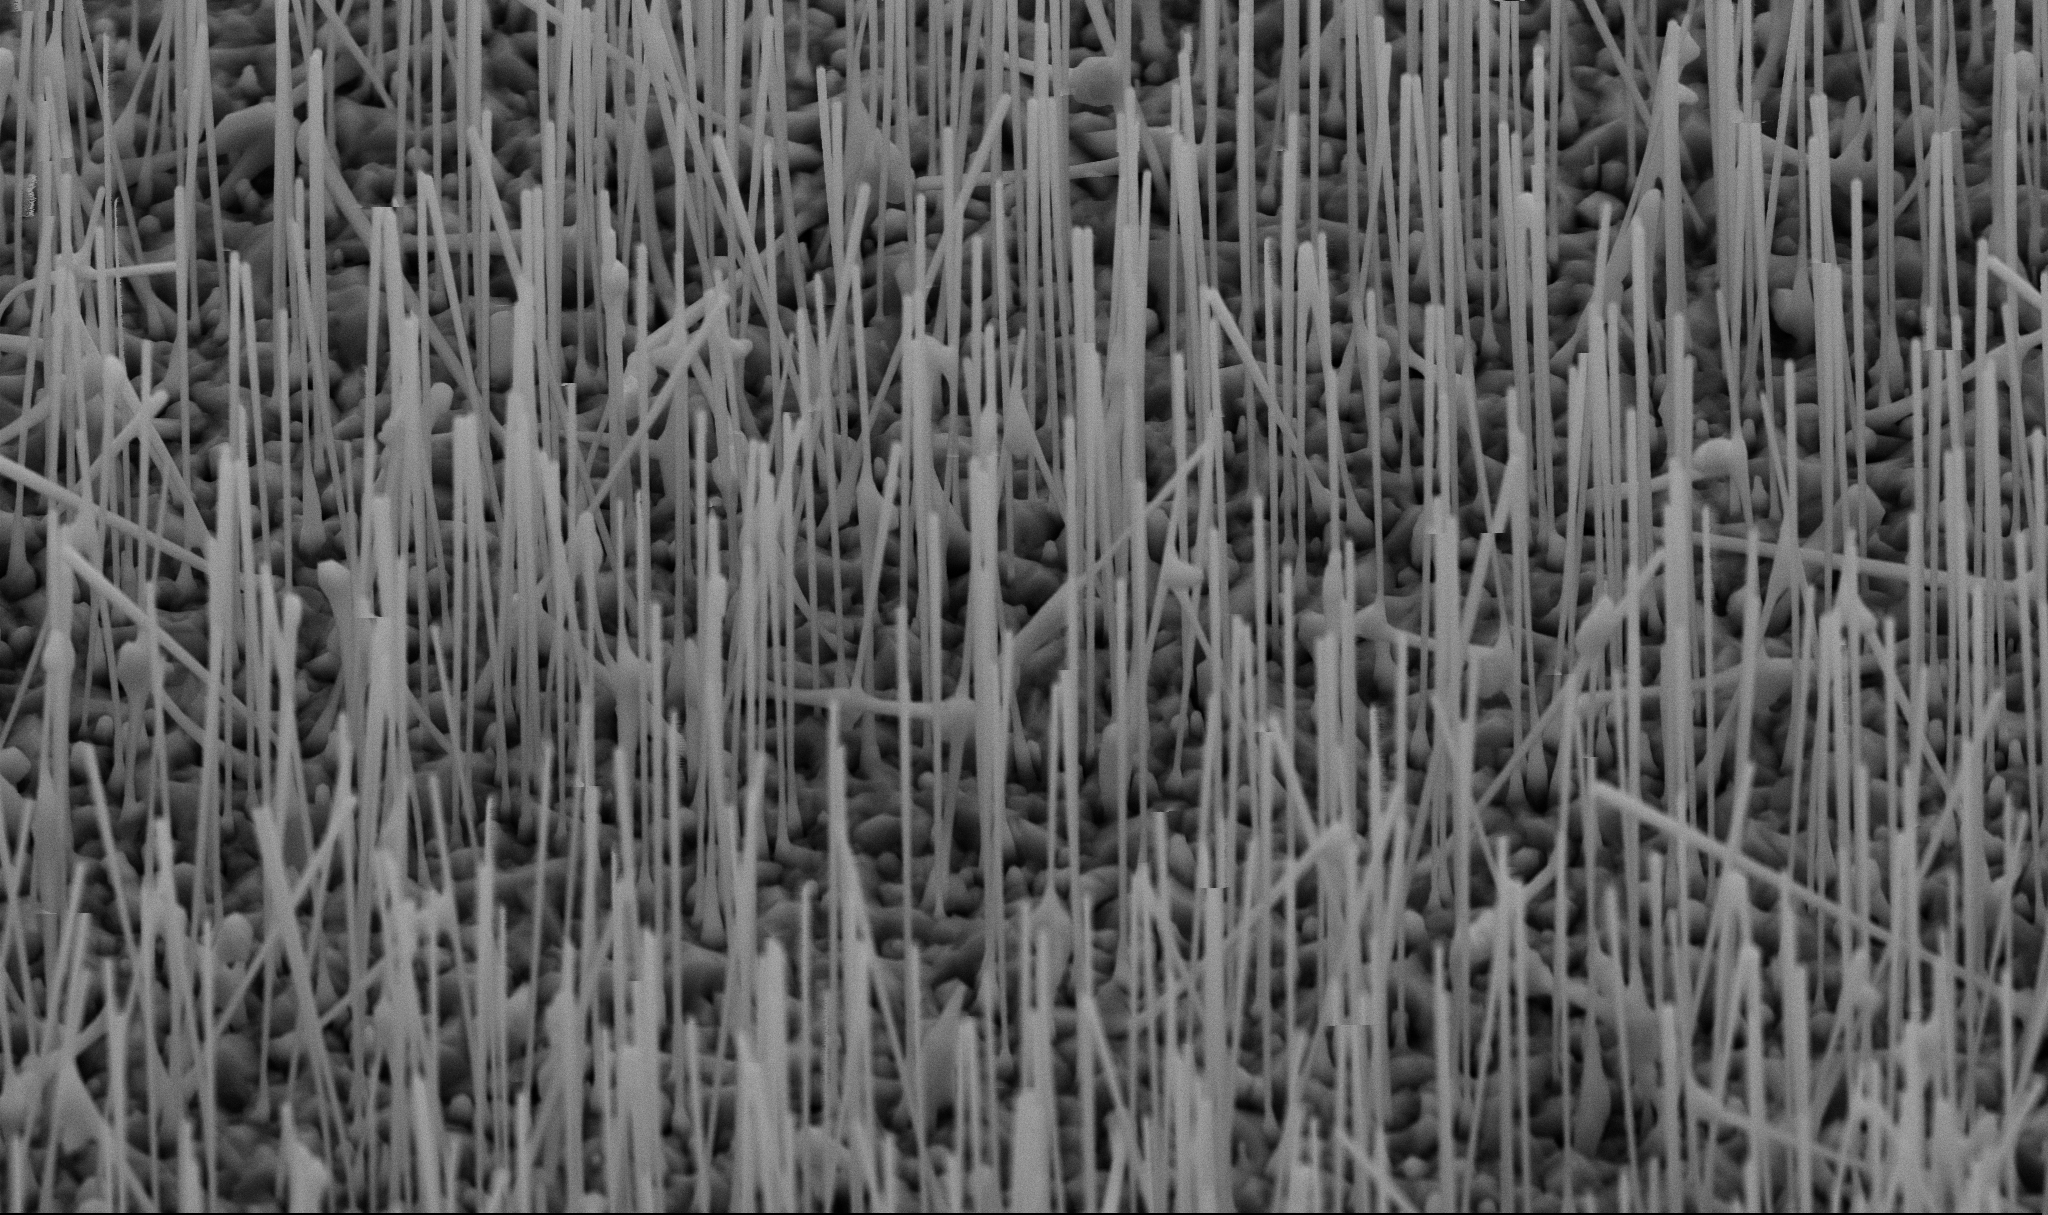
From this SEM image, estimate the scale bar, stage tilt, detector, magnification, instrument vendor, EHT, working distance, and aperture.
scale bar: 2000 nm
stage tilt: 45°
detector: SE2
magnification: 15 K X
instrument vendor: Zeiss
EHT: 10 kV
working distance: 7.2 mm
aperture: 30 µm